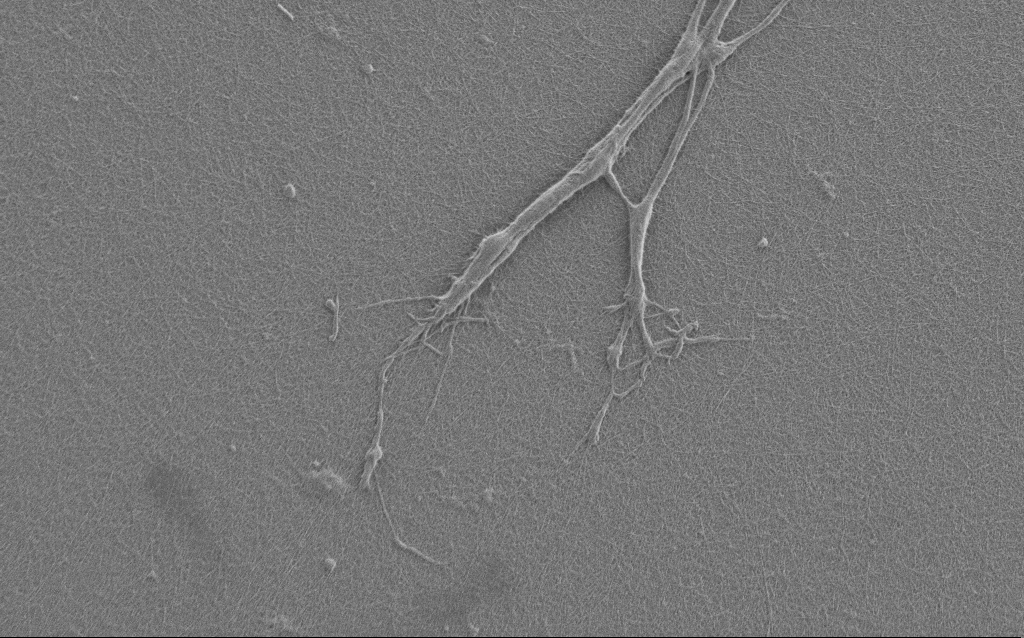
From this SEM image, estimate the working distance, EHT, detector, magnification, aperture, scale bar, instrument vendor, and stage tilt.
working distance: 6 mm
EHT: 1 kV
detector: SE2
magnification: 6 K X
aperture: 30 µm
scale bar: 10000 nm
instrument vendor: Zeiss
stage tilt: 0°